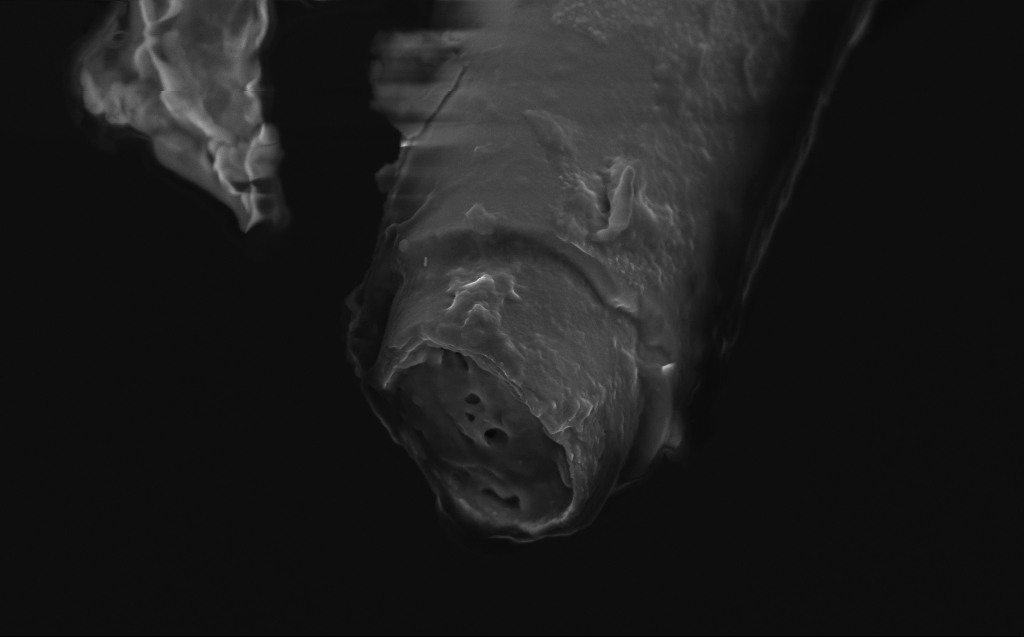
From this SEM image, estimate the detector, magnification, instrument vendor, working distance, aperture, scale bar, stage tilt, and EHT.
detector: InLens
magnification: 36.99 K X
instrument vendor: Zeiss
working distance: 4 mm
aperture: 30 µm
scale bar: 1000 nm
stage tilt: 45°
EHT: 3 kV